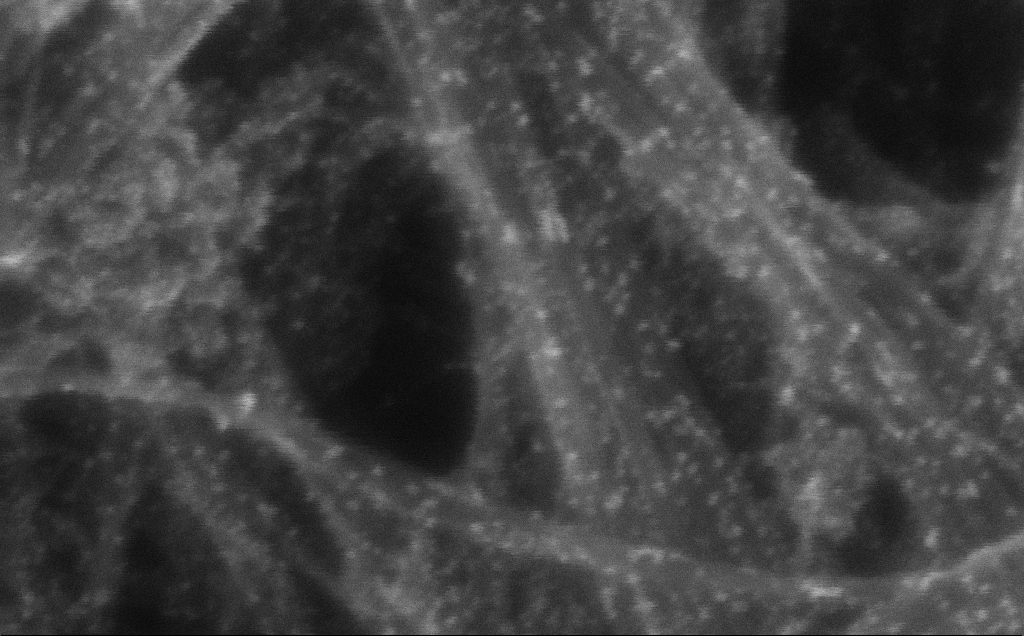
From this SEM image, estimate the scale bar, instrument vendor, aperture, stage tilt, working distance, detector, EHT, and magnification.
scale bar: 20 nm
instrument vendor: Zeiss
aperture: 30 µm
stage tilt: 0°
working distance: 3 mm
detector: InLens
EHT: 10 kV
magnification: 790.09 K X